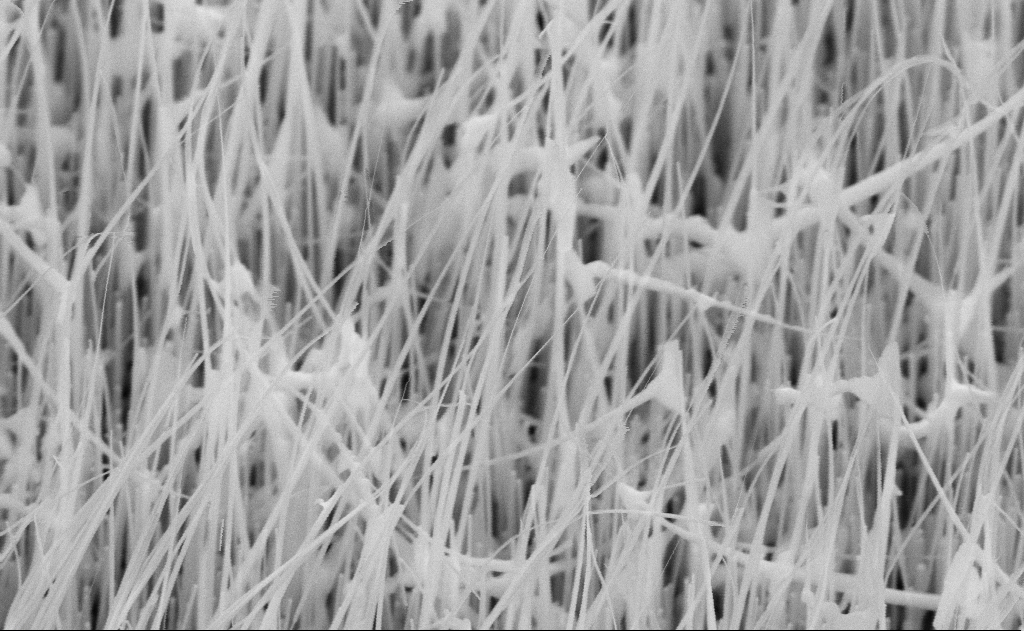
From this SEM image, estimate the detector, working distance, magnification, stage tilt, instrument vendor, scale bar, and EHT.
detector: SE2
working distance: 15 mm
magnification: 60 K X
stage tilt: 45°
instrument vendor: Zeiss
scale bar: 1000 nm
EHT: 10 kV